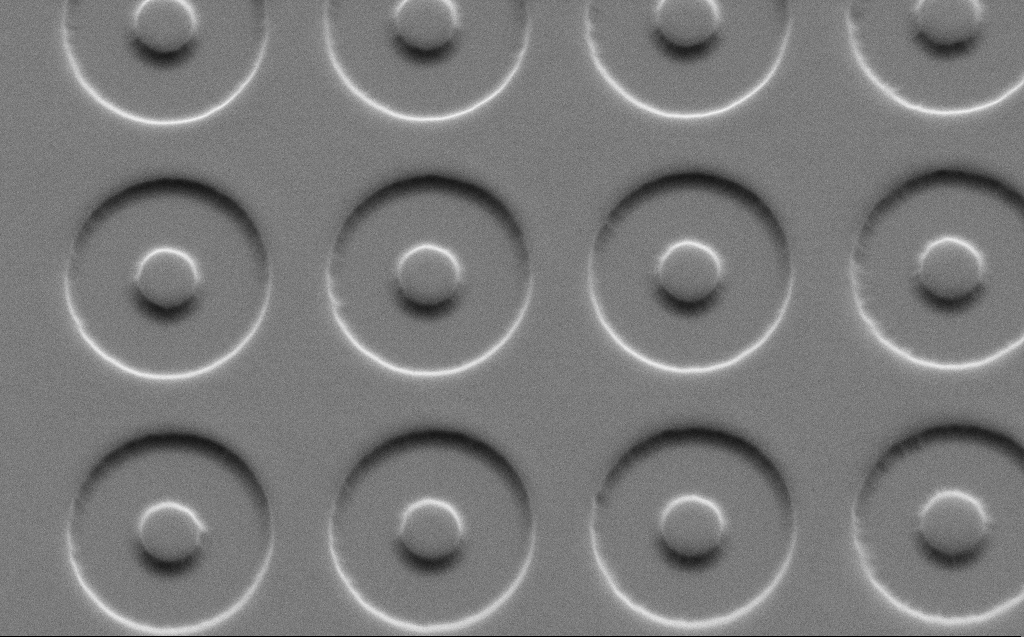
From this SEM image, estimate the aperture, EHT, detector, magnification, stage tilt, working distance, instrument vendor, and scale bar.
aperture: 30 µm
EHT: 3 kV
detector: SE2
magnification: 9.61 K X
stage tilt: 45°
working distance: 6 mm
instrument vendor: Zeiss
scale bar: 2000 nm